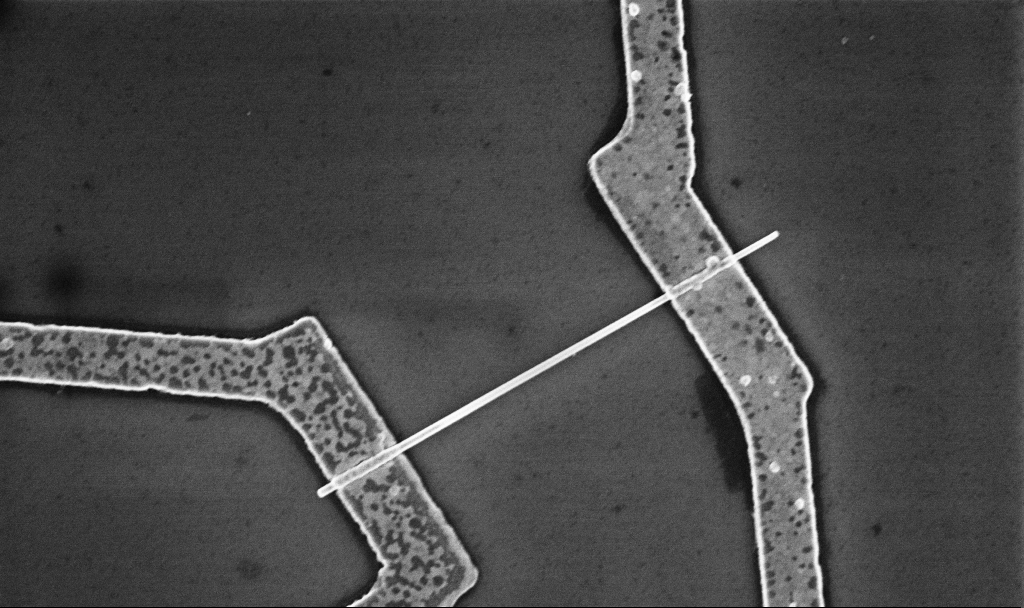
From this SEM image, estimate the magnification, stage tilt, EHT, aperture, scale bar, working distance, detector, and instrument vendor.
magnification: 30 K X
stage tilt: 0°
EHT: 5 kV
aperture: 30 µm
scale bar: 1000 nm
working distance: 8.7 mm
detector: InLens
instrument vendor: Zeiss